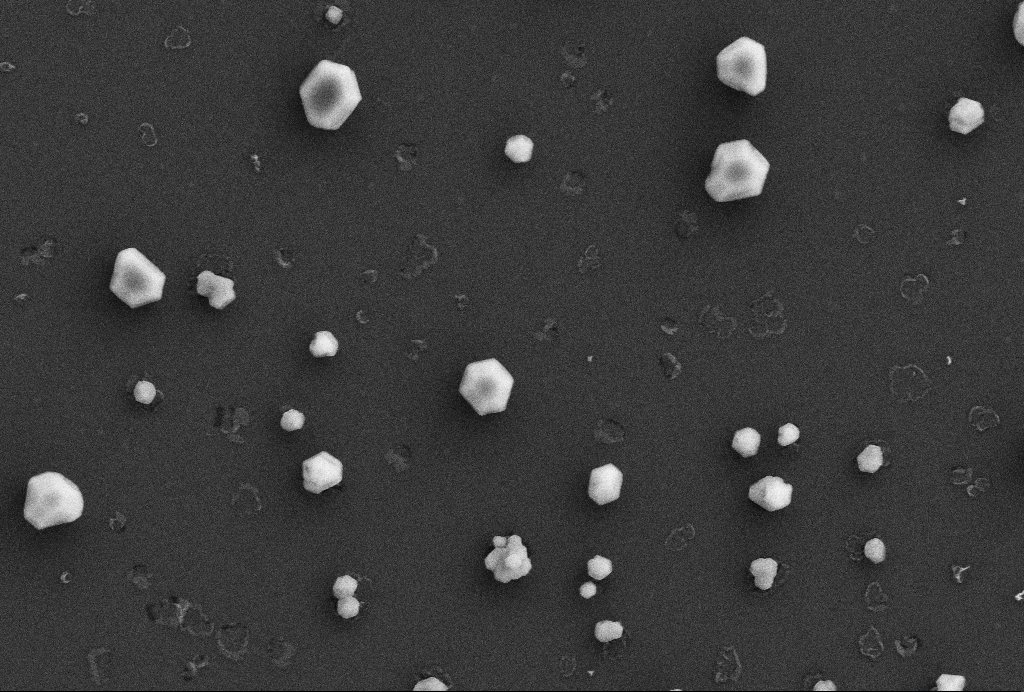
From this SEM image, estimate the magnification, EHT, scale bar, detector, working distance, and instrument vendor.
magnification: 25 K X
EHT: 5 kV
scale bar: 1000 nm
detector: SE2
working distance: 2.6 mm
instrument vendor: Zeiss